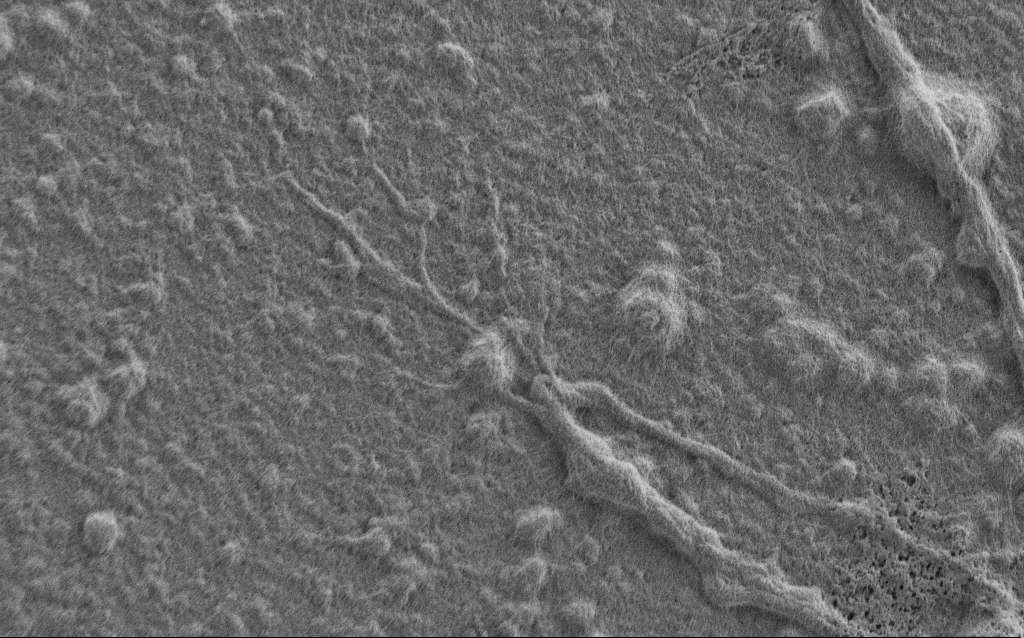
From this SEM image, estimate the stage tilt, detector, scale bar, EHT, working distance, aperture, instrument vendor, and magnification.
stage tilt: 0°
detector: SE2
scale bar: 2000 nm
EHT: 0.9 kV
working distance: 7 mm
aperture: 30 µm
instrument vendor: Zeiss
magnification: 7.5 K X